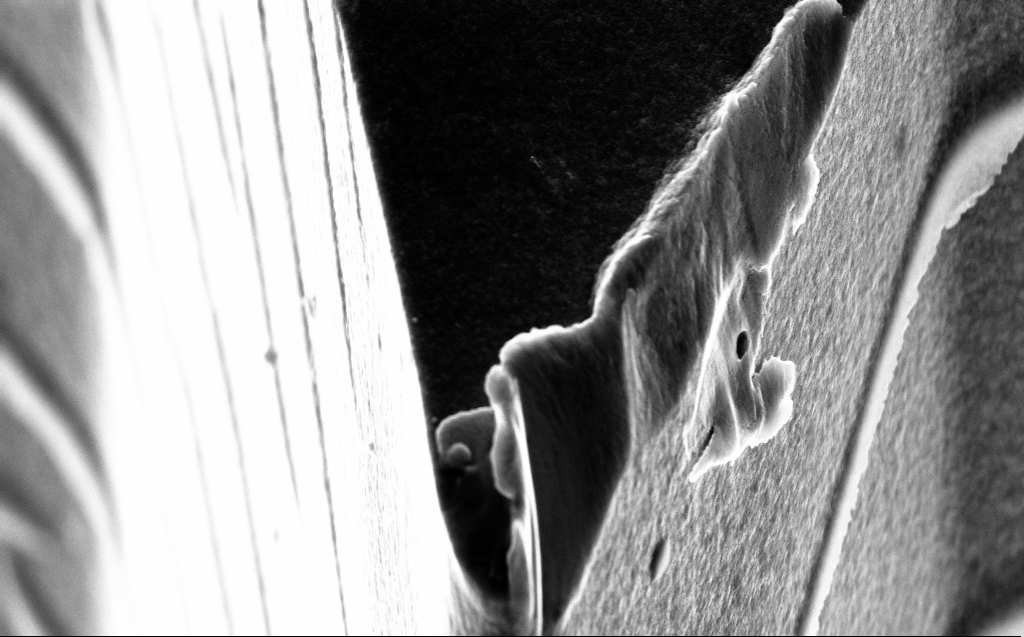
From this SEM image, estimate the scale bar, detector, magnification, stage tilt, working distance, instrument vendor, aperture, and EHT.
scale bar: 2000 nm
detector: InLens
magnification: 25.65 K X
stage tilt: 45°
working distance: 4 mm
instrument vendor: Zeiss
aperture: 30 µm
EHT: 10 kV